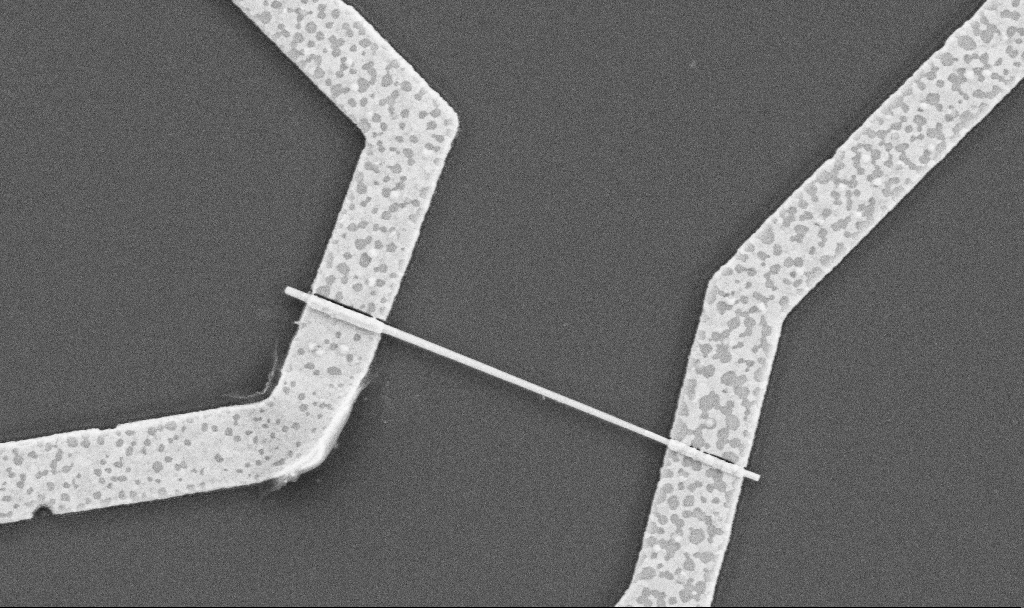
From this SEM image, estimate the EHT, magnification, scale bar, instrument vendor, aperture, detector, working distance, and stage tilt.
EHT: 5 kV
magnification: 30 K X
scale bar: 2000 nm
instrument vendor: Zeiss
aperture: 30 µm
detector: SE2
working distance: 10.5 mm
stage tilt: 0°